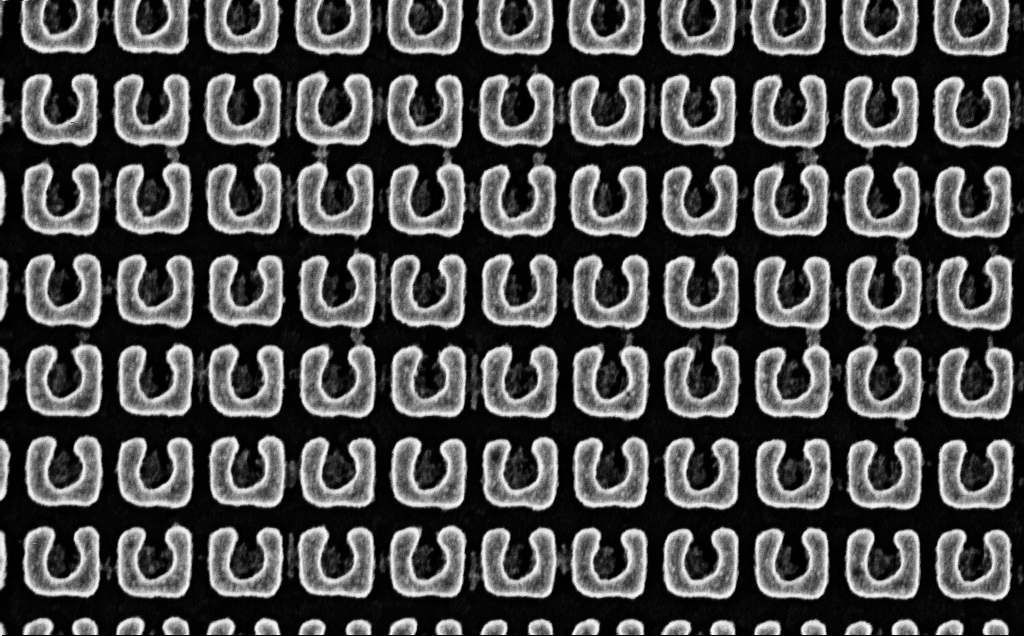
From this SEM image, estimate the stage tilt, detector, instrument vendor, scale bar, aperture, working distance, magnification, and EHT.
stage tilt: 0°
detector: InLens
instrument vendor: Zeiss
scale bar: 200 nm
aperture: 30 µm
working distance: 2.4 mm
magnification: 72.68 K X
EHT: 5 kV